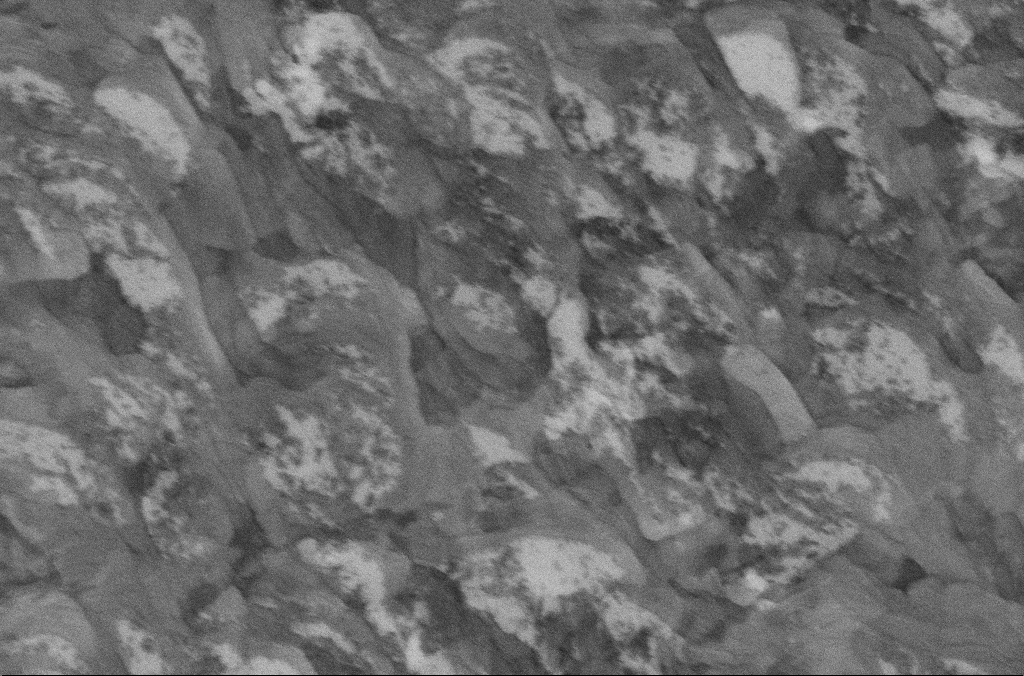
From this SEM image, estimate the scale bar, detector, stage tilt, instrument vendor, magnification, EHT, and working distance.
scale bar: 1000 nm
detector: InLens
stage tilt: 0°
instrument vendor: Zeiss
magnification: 50 K X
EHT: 5 kV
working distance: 6.7 mm